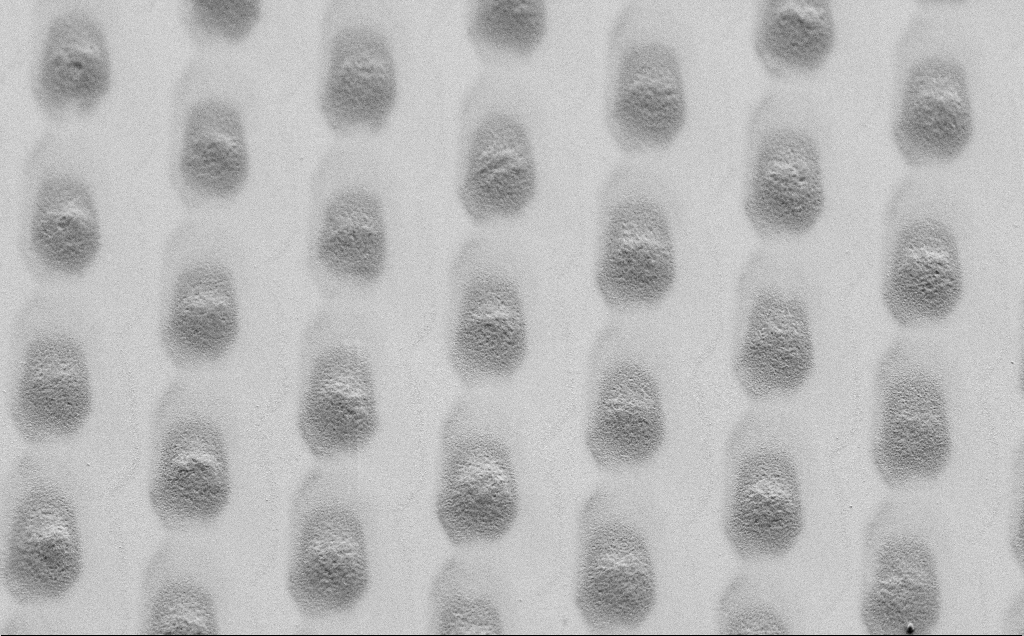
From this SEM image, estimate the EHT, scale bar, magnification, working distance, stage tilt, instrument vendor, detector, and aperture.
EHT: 1.5 kV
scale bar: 10000 nm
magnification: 6.09 K X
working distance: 7 mm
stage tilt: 45°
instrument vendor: Zeiss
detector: SE2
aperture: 30 µm